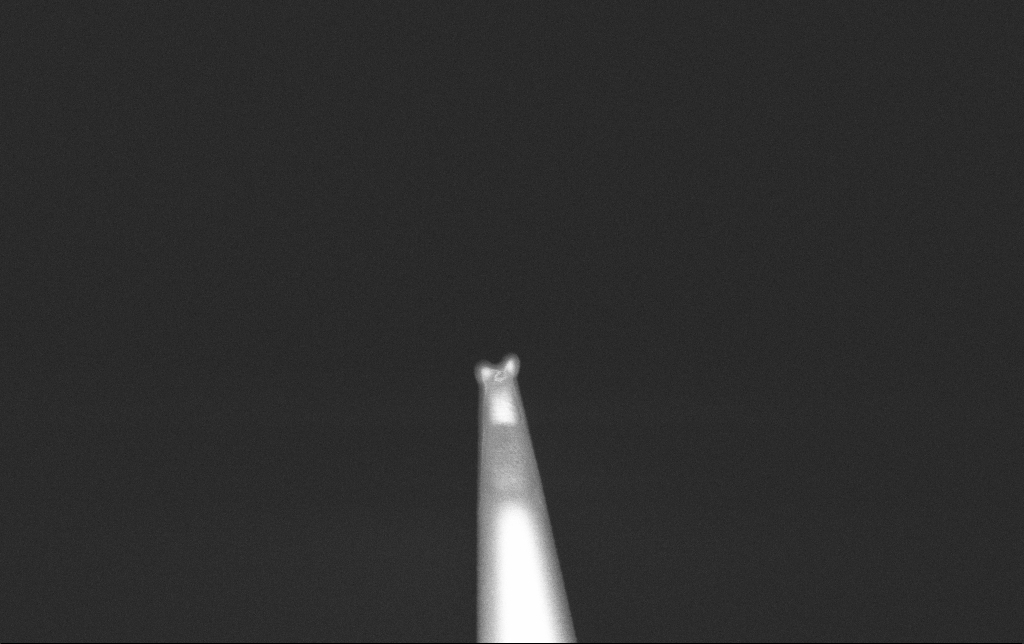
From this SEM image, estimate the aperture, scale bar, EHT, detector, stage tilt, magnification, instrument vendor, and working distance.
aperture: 30 µm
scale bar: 1000 nm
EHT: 2 kV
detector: InLens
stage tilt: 0°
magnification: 50 K X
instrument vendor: Zeiss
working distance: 6.6 mm